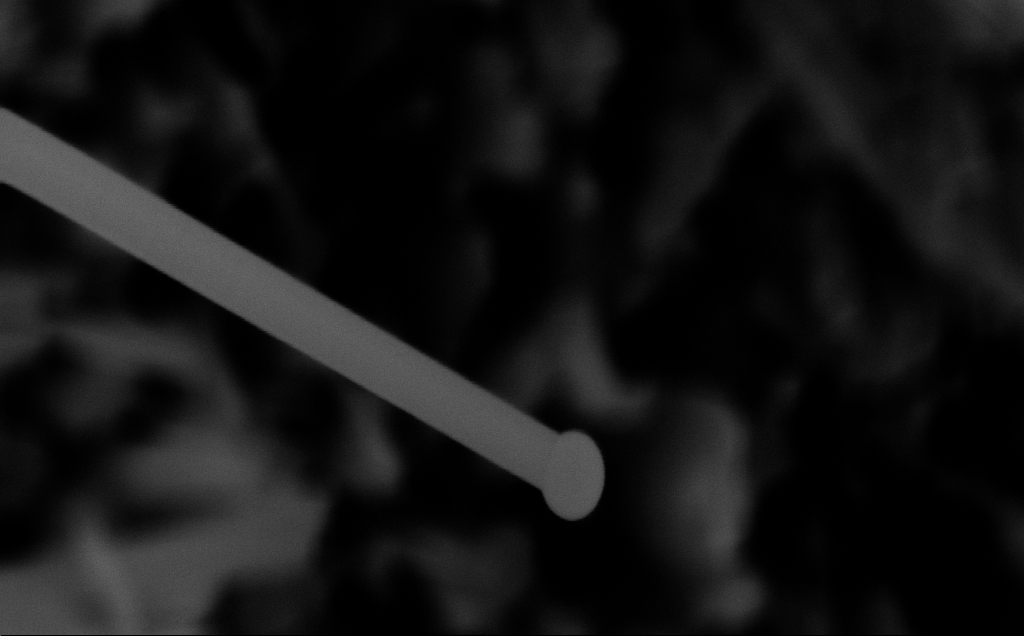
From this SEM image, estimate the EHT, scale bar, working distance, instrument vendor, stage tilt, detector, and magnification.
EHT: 10 kV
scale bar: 200 nm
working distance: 6 mm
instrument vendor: Zeiss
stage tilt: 0°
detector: InLens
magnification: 215.05 K X